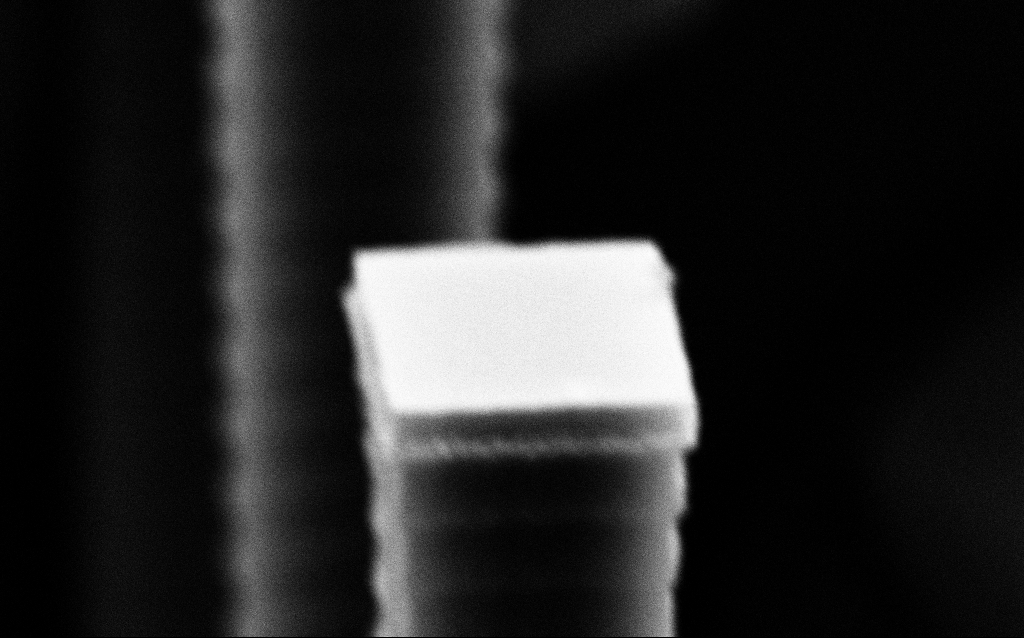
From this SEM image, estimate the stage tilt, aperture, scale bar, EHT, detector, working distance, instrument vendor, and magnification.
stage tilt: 70°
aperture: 30 µm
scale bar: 1000 nm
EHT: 8 kV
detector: SE2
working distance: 6.5 mm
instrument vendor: Zeiss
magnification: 63.87 K X